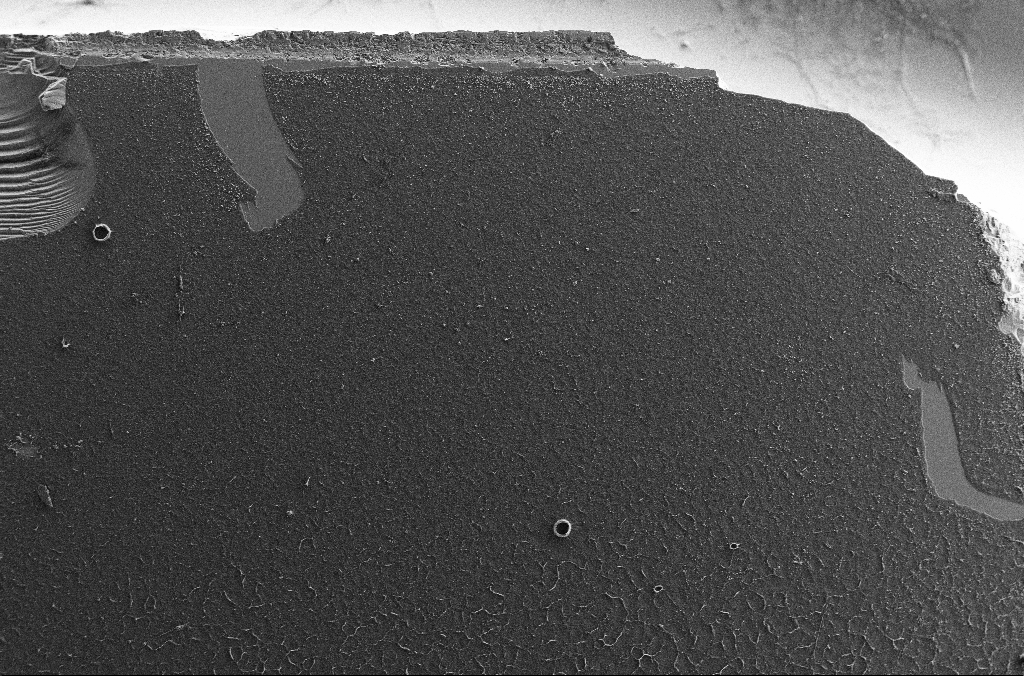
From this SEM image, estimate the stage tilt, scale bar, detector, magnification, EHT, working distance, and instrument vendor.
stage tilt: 0°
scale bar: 100000 nm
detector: SE2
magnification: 0.15 K X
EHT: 7 kV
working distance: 2.9 mm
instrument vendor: Zeiss